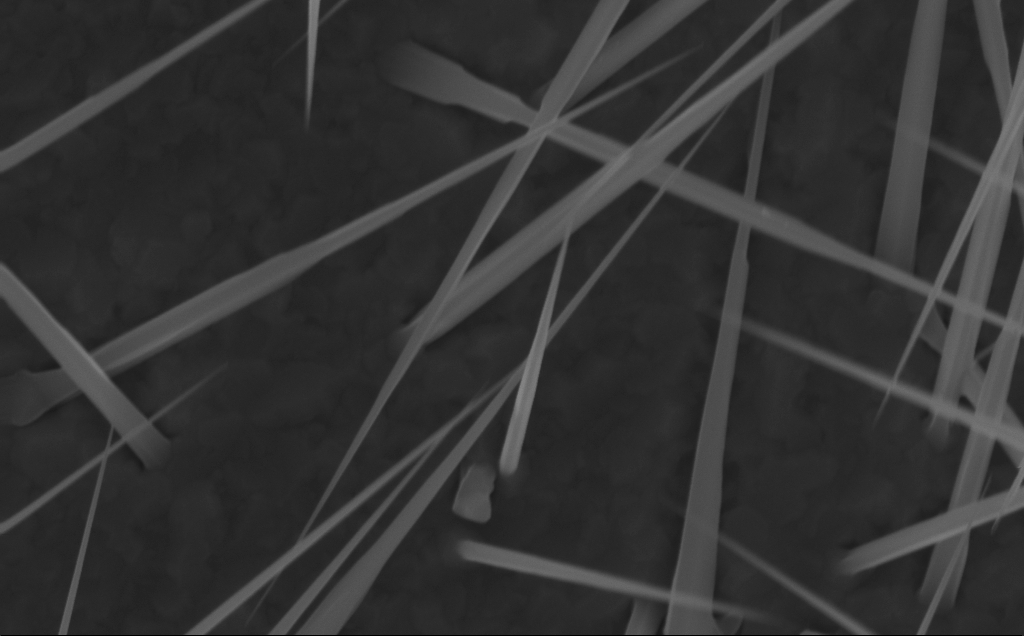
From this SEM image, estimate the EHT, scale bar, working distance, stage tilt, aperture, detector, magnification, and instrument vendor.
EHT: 10 kV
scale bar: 200 nm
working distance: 6 mm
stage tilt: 0°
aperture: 30 µm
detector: InLens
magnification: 80 K X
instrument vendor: Zeiss